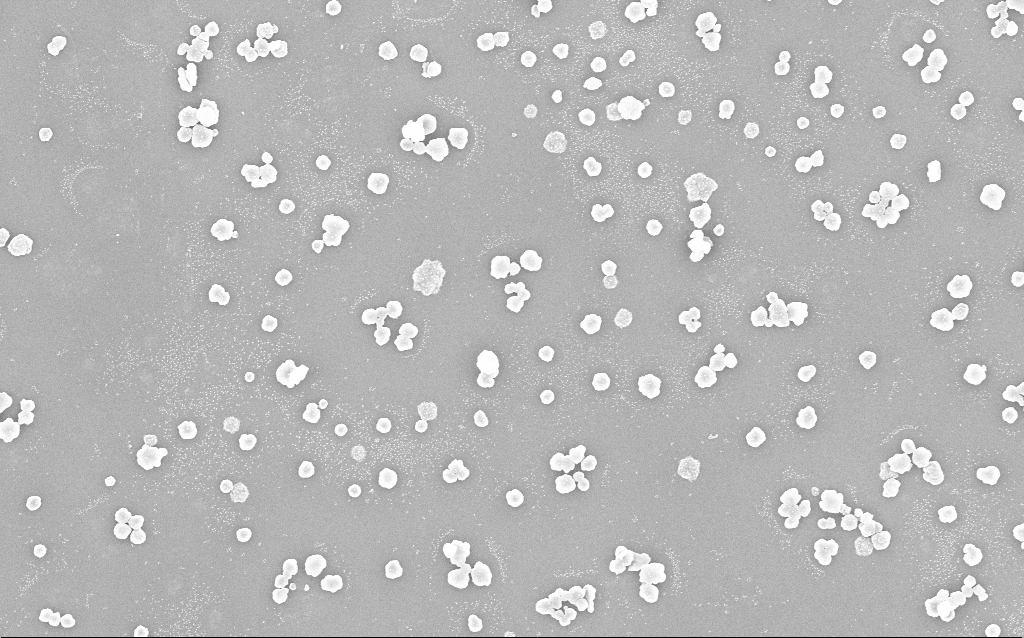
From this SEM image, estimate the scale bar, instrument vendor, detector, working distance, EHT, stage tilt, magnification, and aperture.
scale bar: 2000 nm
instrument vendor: Zeiss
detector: InLens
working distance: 1.4 mm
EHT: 20 kV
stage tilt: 0°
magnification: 10 K X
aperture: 30 µm